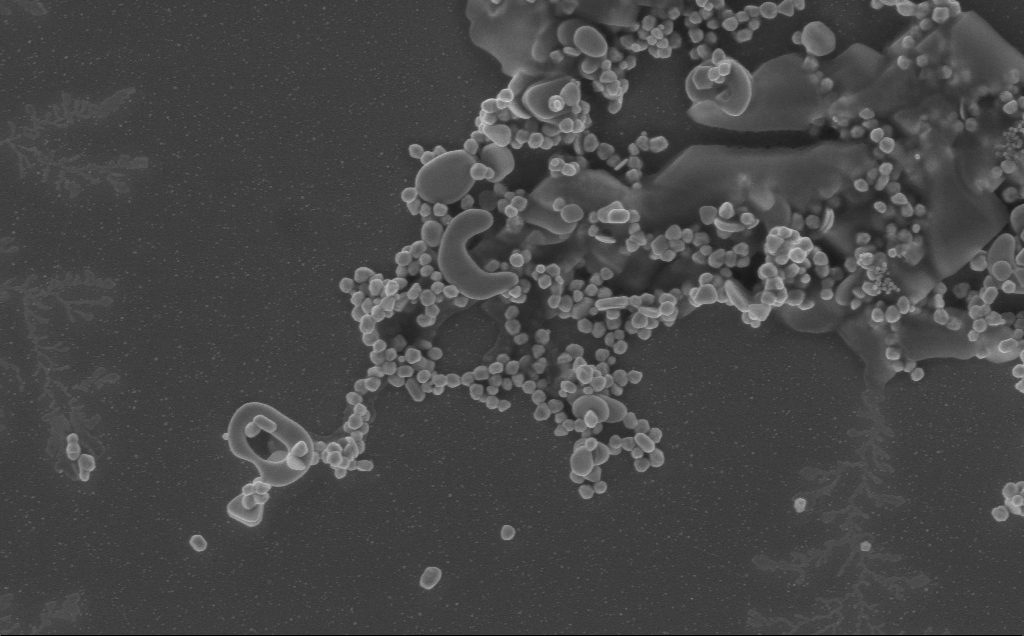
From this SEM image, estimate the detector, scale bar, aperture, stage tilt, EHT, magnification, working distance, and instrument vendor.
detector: InLens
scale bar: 1000 nm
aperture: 30 µm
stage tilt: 0°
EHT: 5 kV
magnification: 40 K X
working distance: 3 mm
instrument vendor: Zeiss